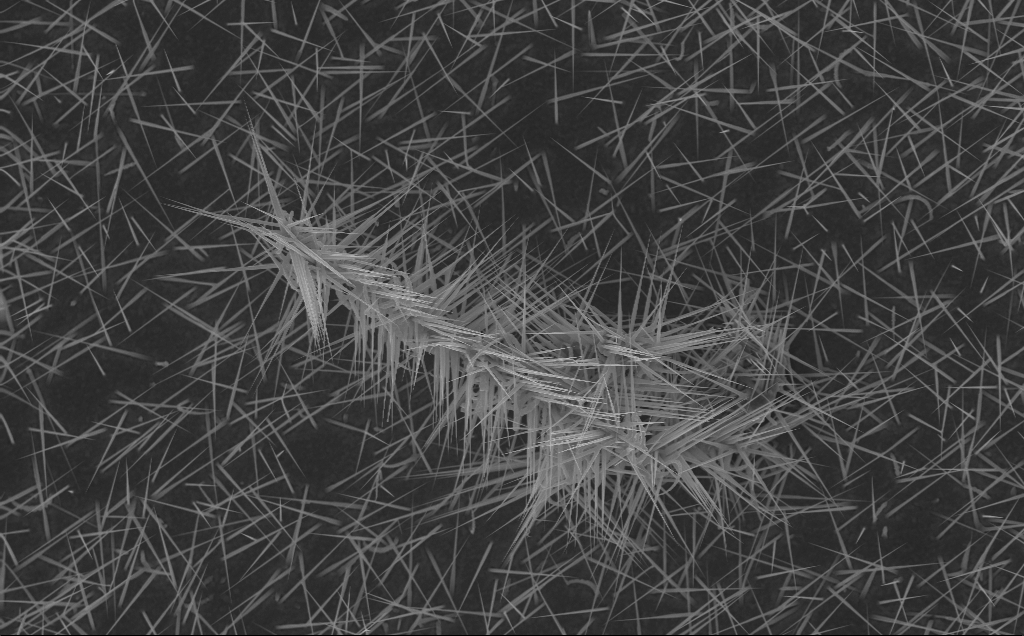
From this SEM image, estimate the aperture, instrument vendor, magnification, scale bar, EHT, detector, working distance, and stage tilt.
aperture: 30 µm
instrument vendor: Zeiss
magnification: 9.41 K X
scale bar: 2000 nm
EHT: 10 kV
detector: InLens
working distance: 6 mm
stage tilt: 0°